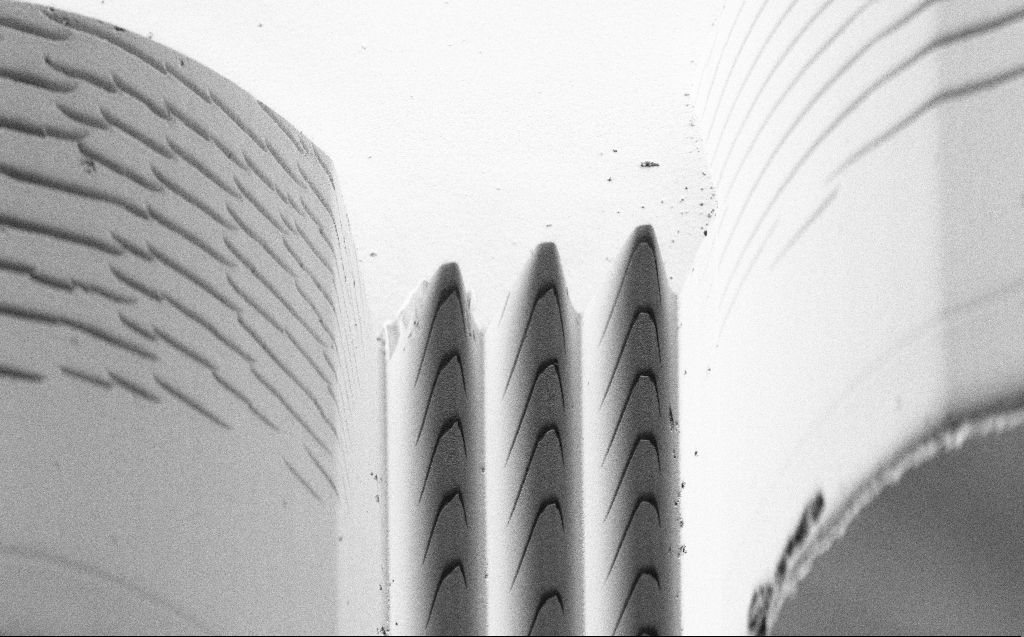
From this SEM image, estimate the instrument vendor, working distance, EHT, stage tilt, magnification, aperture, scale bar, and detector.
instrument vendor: Zeiss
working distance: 4 mm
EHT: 3 kV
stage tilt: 45°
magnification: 3.25 K X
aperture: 30 µm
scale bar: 20000 nm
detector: SE2